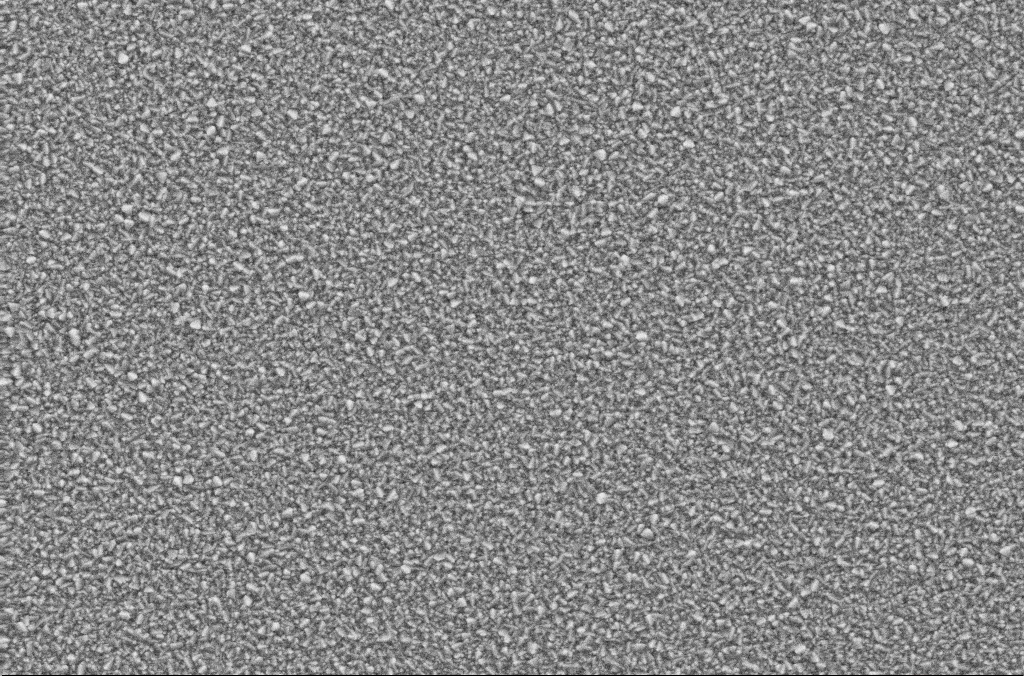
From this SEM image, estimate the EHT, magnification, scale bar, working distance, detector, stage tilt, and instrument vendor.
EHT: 10 kV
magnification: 10 K X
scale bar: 2000 nm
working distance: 1.9 mm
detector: SE2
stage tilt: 0°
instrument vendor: Zeiss